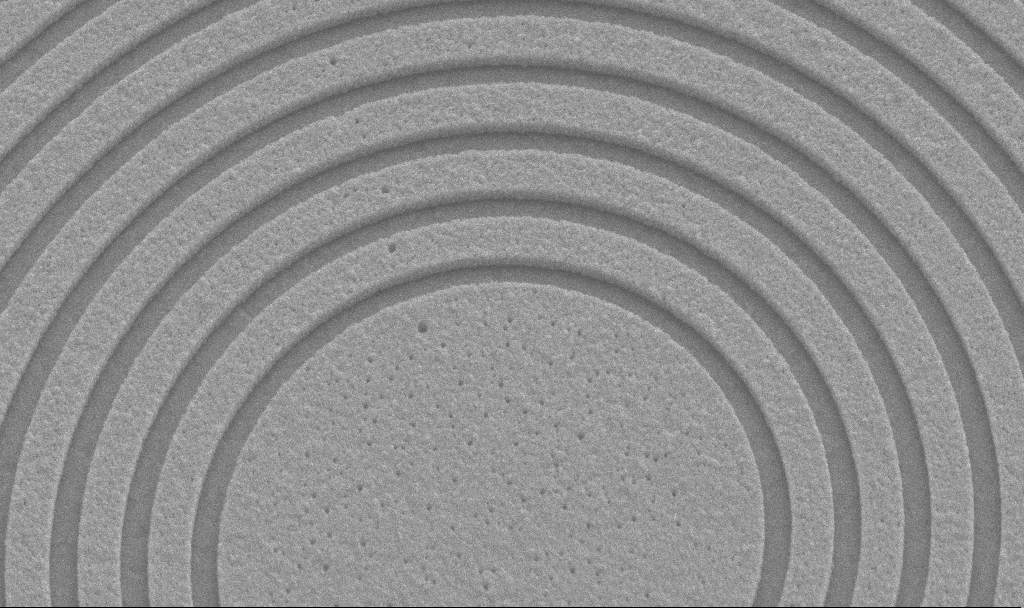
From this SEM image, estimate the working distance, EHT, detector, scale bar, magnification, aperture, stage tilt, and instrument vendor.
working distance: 9.8 mm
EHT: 5 kV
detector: SE2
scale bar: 1000 nm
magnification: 40.08 K X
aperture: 30 µm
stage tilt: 45°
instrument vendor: Zeiss